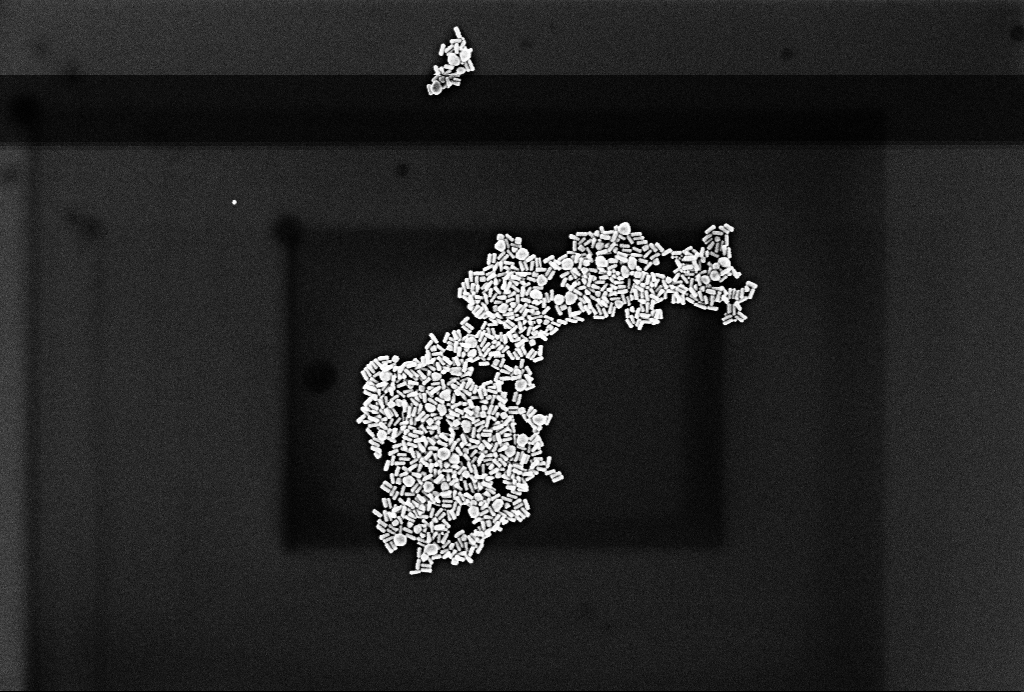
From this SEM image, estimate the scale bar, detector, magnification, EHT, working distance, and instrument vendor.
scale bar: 1000 nm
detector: InLens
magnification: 60 K X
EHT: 2 kV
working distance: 3.3 mm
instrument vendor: Zeiss